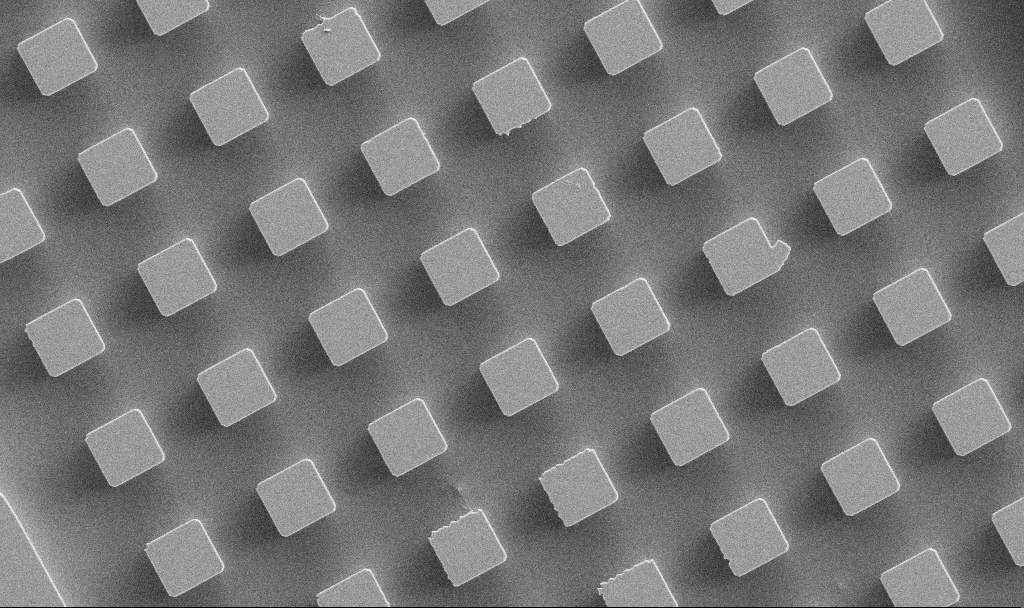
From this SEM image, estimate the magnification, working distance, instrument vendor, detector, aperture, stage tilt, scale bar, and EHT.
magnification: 2.34 K X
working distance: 5.6 mm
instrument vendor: Zeiss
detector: SE2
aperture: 30 µm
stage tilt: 0°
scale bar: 10000 nm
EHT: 5 kV